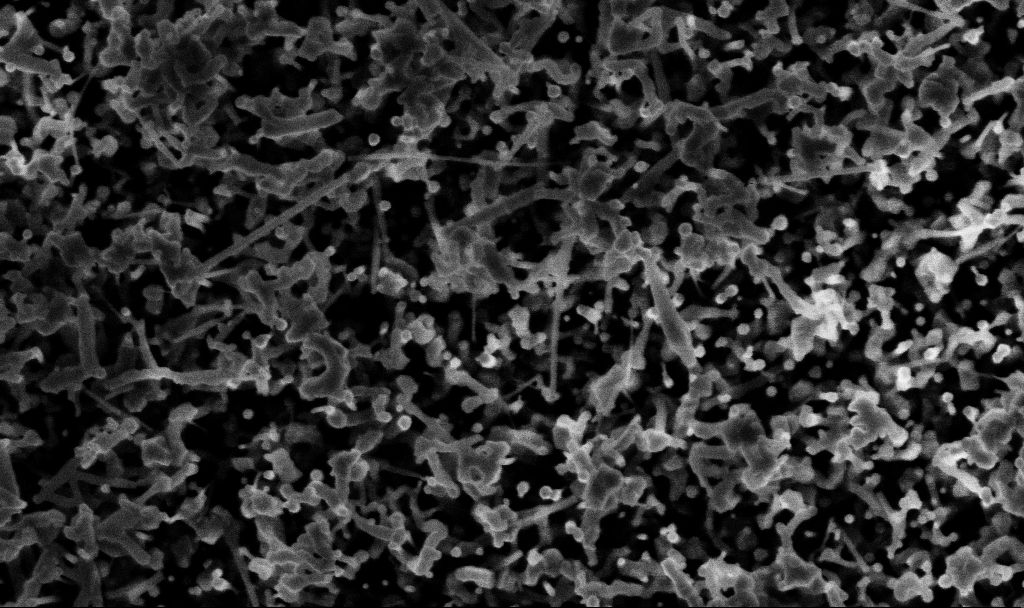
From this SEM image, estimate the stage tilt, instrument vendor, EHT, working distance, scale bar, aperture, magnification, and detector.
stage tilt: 0°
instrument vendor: Zeiss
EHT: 3 kV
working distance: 3.1 mm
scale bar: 200 nm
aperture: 30 µm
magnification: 88.79 K X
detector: InLens